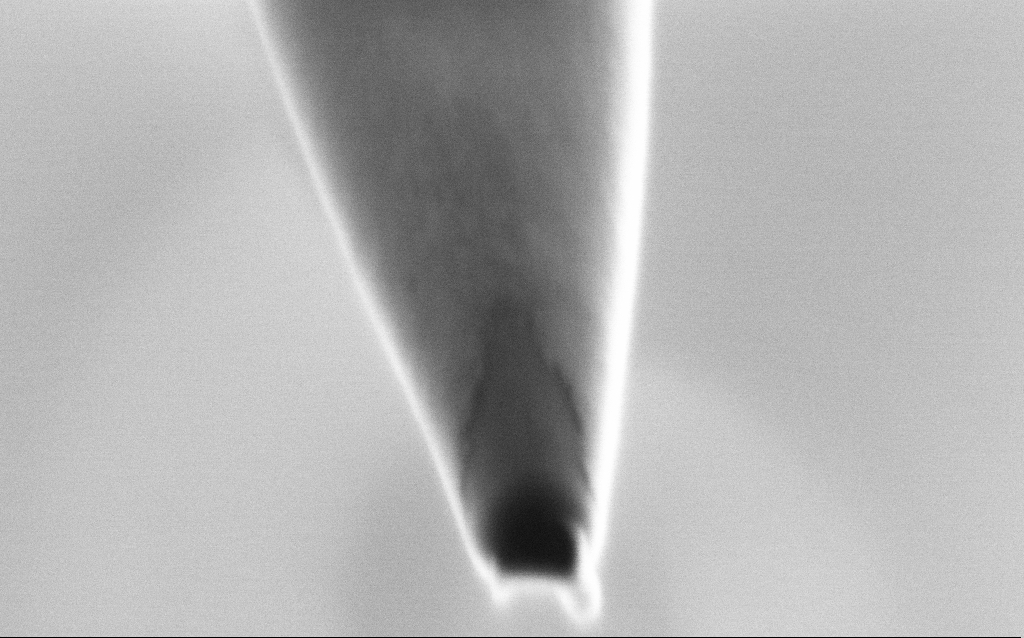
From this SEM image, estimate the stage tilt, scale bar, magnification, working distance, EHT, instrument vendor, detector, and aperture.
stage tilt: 45°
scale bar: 200 nm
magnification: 250 K X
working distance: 6 mm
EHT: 1 kV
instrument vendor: Zeiss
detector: SE2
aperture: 30 µm